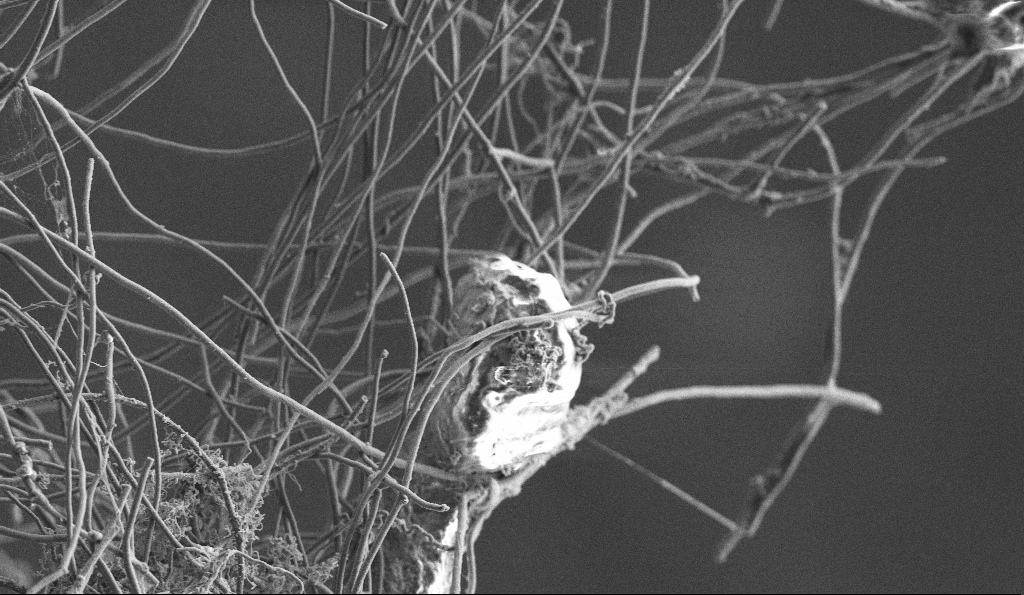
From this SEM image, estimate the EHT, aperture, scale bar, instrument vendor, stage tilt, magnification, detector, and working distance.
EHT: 3 kV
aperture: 30 µm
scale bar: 10000 nm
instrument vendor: Zeiss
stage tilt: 0°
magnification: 5 K X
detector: SE2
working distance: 5.9 mm